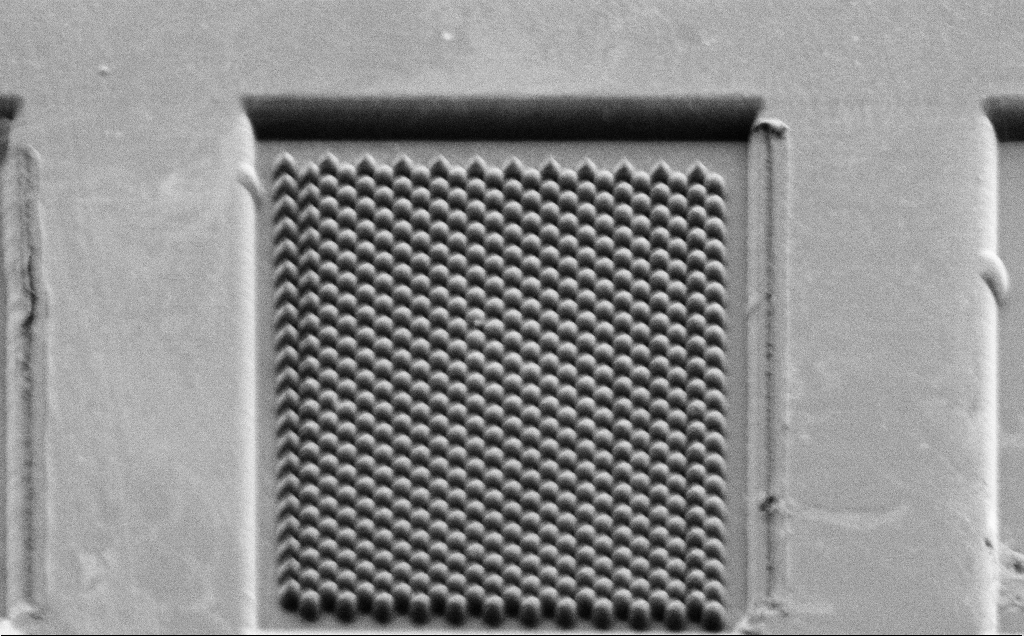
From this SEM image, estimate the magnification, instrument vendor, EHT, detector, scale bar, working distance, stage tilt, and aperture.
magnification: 1.96 K X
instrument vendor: Zeiss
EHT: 1.2 kV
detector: SE2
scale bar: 10000 nm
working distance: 8 mm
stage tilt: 45°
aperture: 30 µm